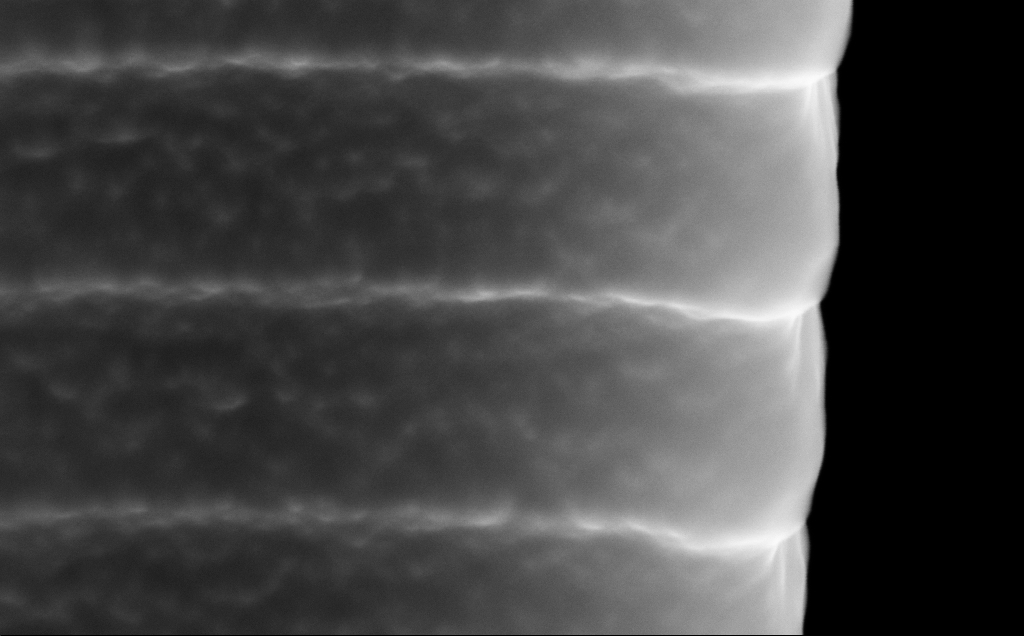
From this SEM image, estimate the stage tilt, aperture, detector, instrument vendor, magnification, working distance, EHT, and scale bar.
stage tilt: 45°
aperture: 30 µm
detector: InLens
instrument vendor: Zeiss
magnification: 207.32 K X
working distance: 5 mm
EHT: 10 kV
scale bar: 100 nm